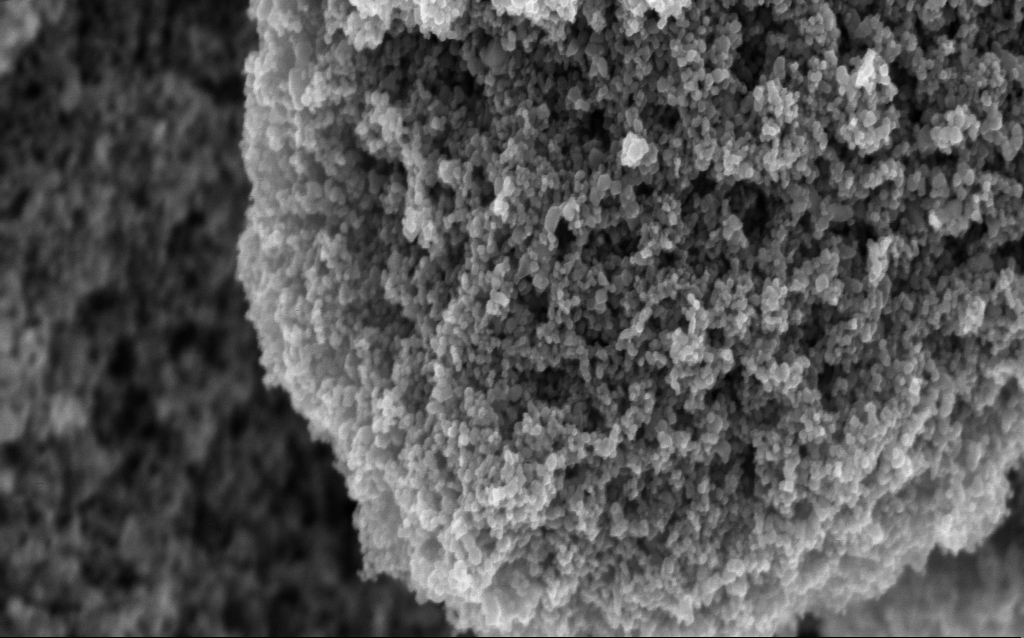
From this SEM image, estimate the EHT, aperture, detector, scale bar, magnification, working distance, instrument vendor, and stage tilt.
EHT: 5 kV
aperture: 30 µm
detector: InLens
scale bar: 200 nm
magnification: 103.76 K X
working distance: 4.6 mm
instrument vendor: Zeiss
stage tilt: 0°